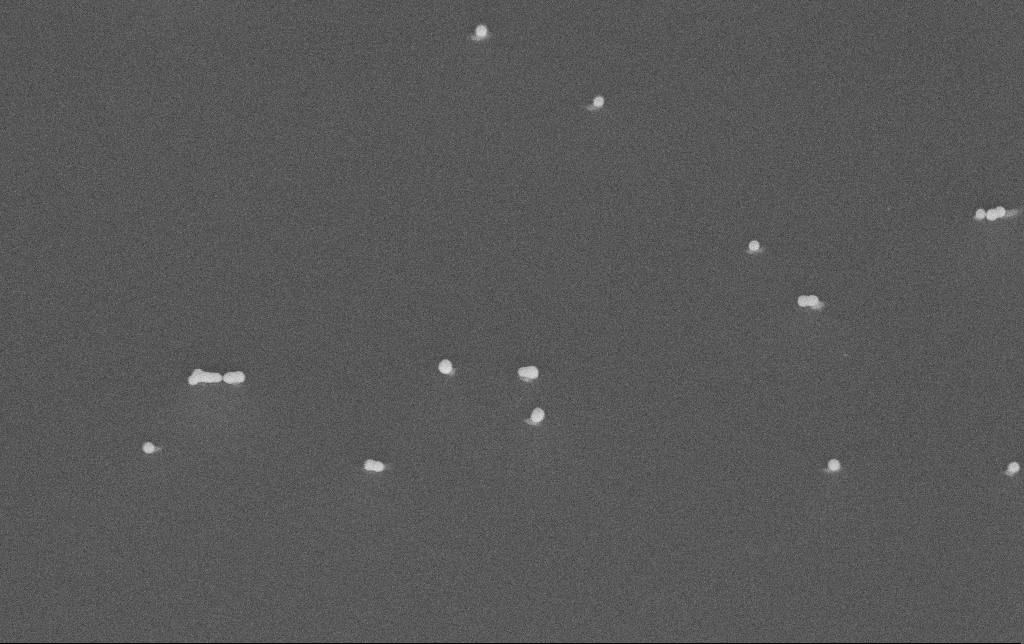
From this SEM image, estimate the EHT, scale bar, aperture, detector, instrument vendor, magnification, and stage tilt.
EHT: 10 kV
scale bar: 1000 nm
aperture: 30 µm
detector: InLens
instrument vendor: Zeiss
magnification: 71.95 K X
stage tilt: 60°